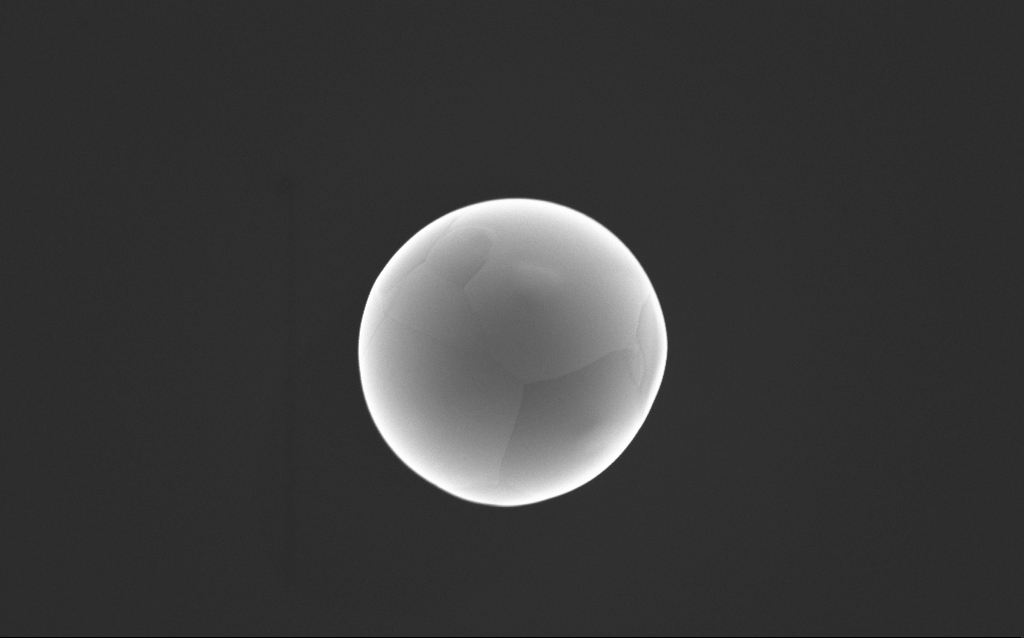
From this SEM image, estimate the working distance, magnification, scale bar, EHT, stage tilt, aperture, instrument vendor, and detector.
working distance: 3 mm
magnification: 69.78 K X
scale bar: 1000 nm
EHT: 10 kV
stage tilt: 0°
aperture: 30 µm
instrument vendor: Zeiss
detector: InLens